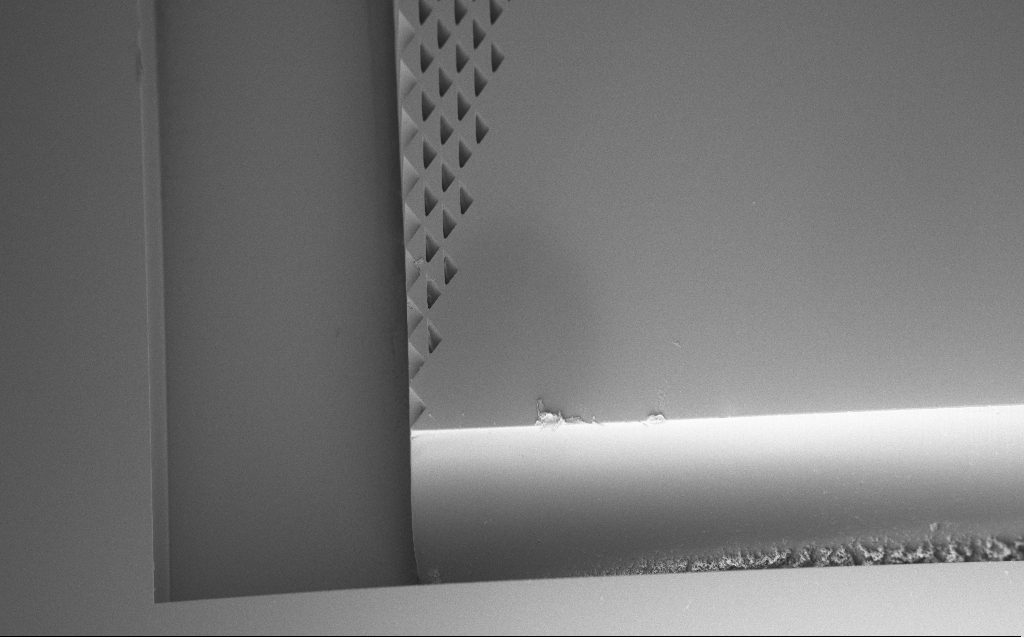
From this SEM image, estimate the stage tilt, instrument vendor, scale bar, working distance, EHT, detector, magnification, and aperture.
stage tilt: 45°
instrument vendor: Zeiss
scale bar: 100000 nm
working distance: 7 mm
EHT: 3 kV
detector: InLens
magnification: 0.188 K X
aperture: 30 µm